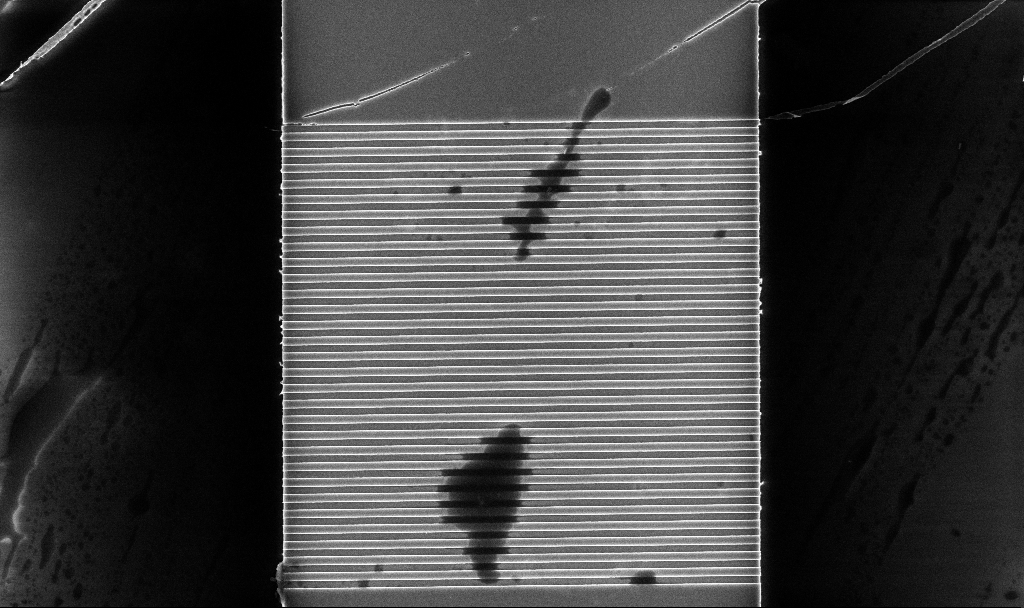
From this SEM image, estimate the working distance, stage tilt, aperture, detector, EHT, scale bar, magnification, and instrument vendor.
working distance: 5.2 mm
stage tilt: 0°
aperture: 30 µm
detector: InLens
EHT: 5 kV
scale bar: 2000 nm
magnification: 8.87 K X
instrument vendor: Zeiss